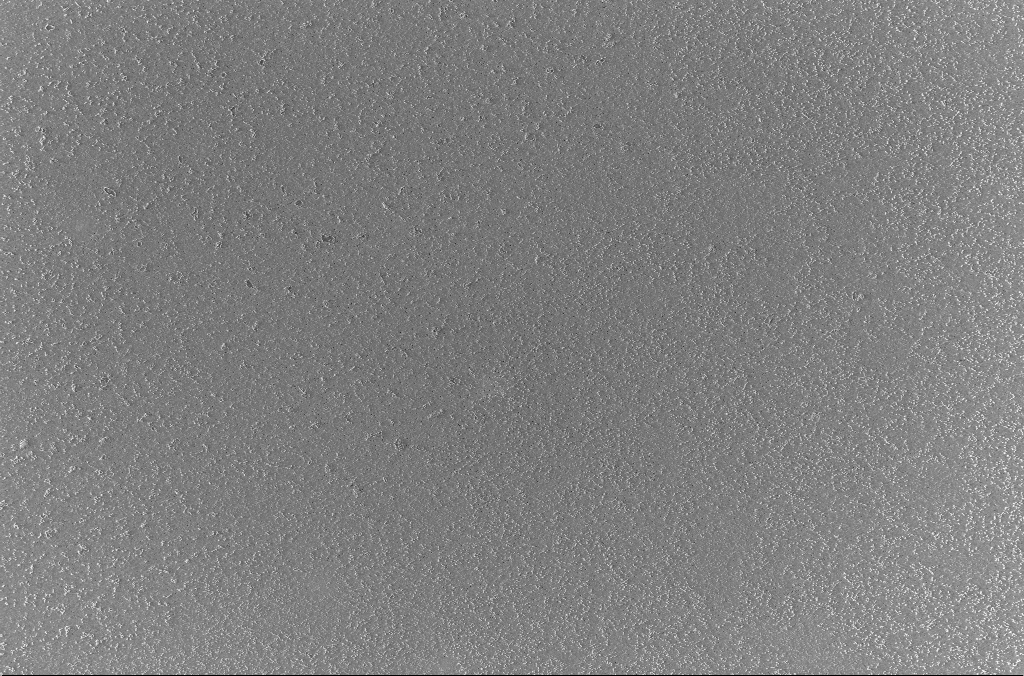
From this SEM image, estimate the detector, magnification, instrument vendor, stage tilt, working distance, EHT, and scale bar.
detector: InLens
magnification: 1 K X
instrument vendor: Zeiss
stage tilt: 0°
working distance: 3 mm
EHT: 2 kV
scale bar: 20000 nm